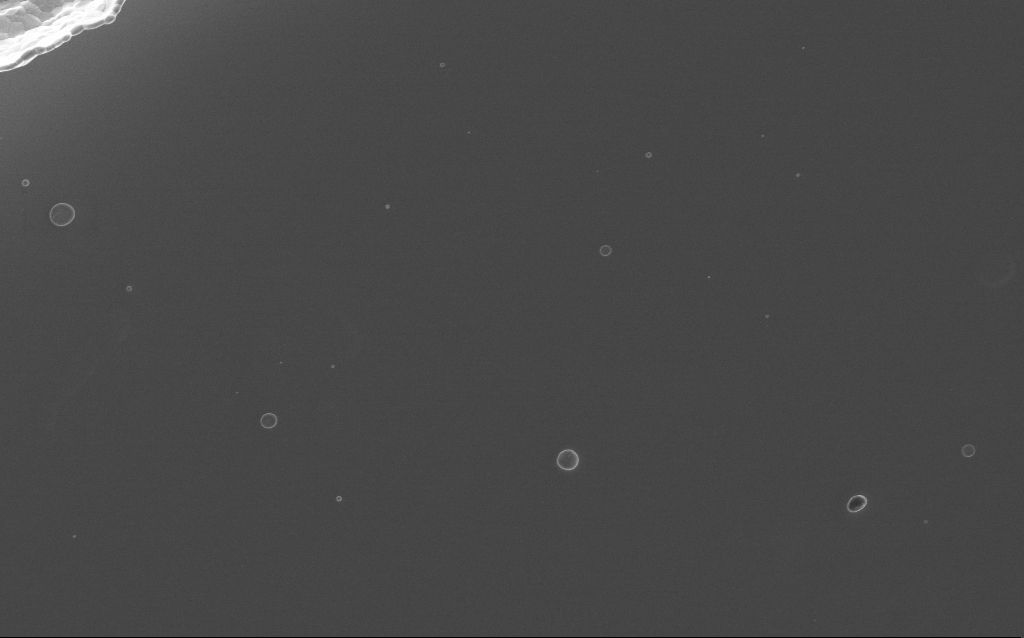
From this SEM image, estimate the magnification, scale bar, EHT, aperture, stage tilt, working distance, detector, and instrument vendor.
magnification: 8.54 K X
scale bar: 2000 nm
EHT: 5 kV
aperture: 30 µm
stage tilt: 0°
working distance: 2 mm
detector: InLens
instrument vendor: Zeiss